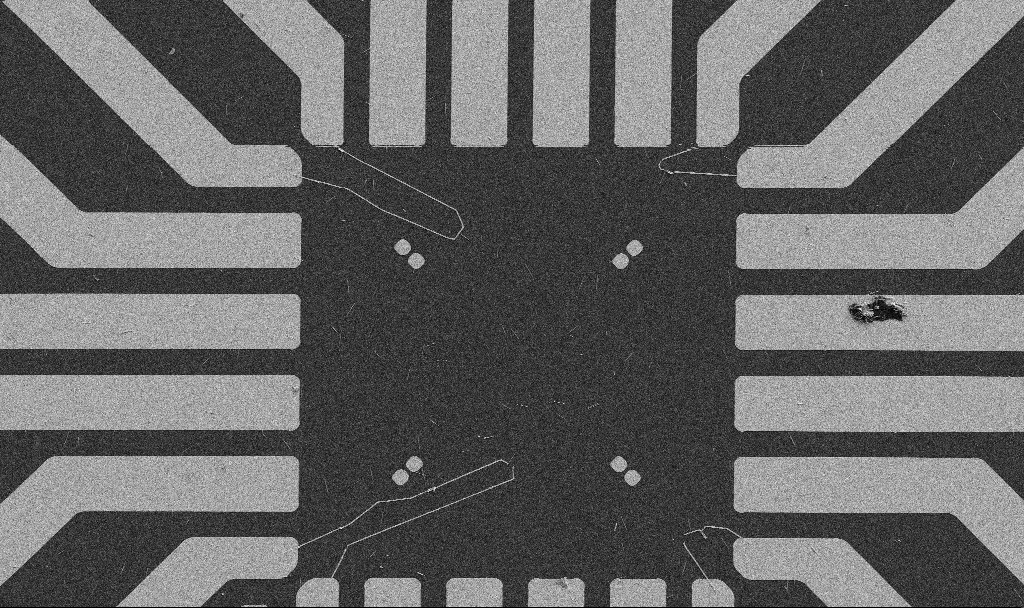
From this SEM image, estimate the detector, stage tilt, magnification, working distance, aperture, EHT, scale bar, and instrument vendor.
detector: SE2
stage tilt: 0°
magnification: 1 K X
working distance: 10.7 mm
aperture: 30 µm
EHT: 5 kV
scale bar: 20000 nm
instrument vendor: Zeiss